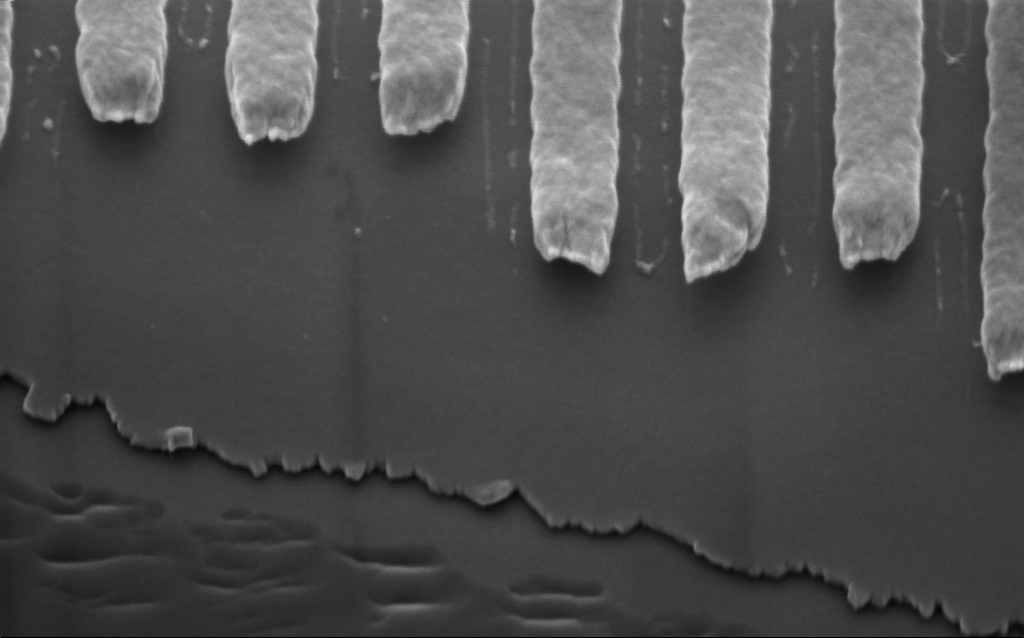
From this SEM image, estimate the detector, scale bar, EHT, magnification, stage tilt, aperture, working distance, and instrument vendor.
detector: InLens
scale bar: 200 nm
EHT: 2 kV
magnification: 111.89 K X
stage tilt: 45°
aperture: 30 µm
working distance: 3.4 mm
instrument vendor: Zeiss